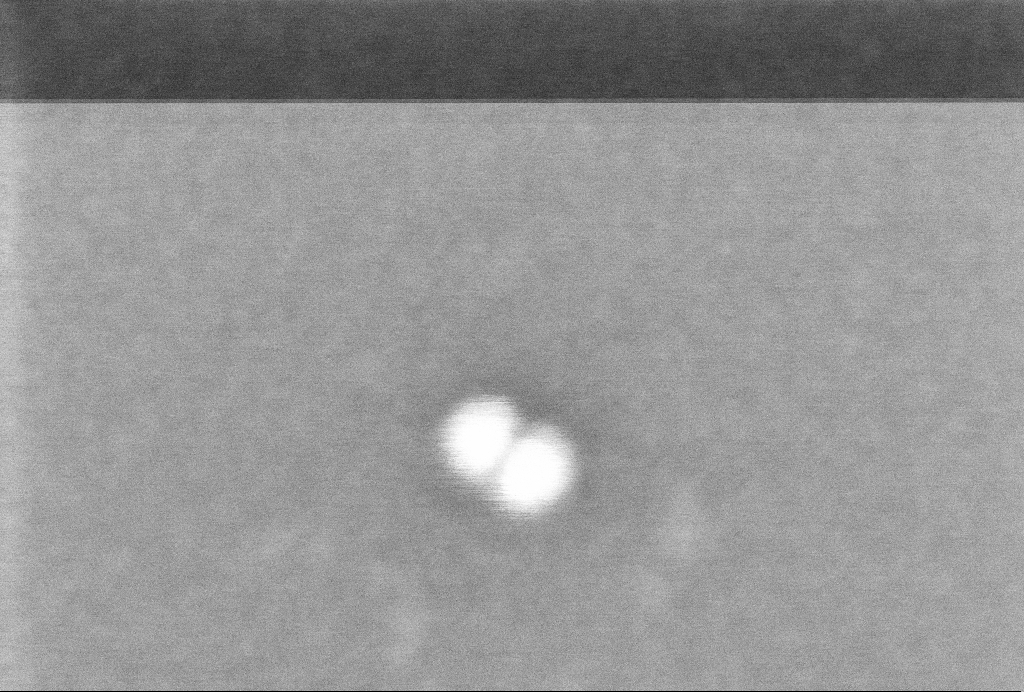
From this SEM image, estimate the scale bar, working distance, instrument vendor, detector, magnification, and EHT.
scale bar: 20 nm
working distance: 3.3 mm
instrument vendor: Zeiss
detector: InLens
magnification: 872.72 K X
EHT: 2 kV